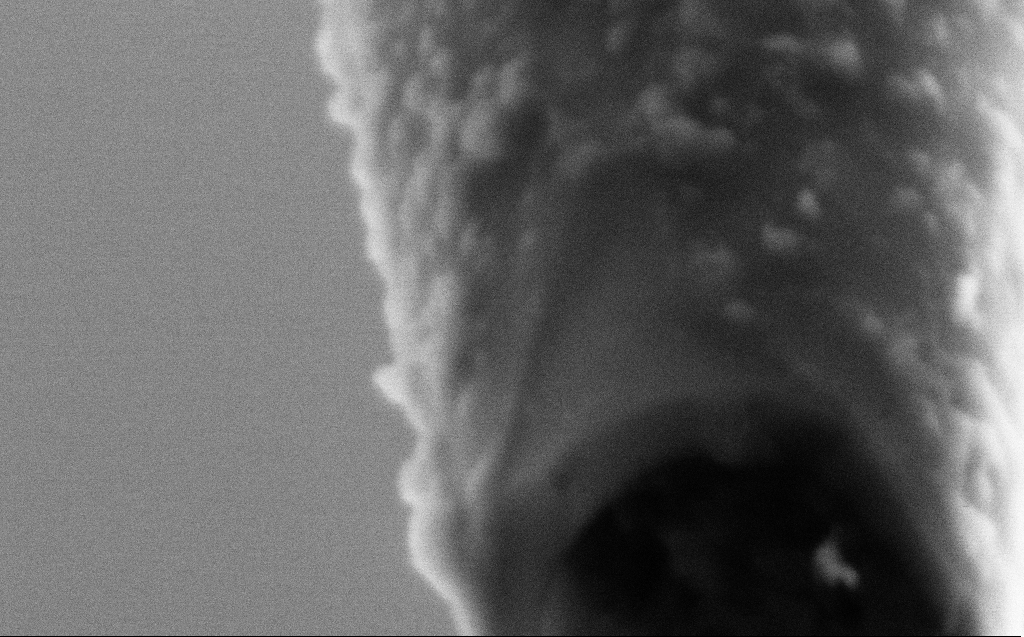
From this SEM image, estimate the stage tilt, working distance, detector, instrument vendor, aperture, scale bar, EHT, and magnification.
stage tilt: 45°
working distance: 4 mm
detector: SE2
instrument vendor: Zeiss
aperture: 30 µm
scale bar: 100 nm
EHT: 2 kV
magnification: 400 K X